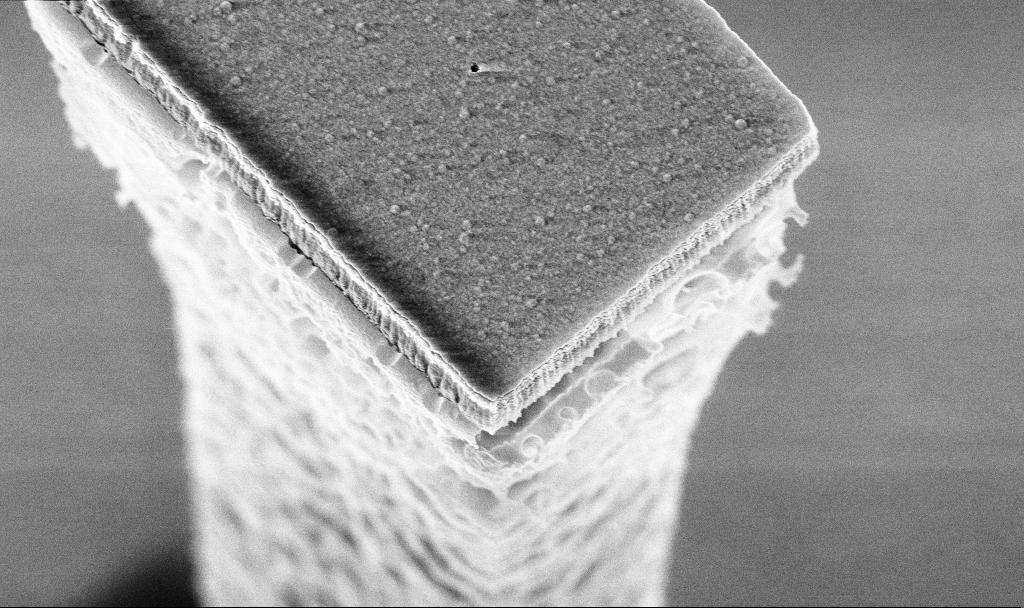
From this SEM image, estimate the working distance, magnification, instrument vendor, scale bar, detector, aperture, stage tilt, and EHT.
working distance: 3.3 mm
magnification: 55.68 K X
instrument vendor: Zeiss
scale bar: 1000 nm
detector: InLens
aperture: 30 µm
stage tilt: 20°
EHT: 5 kV